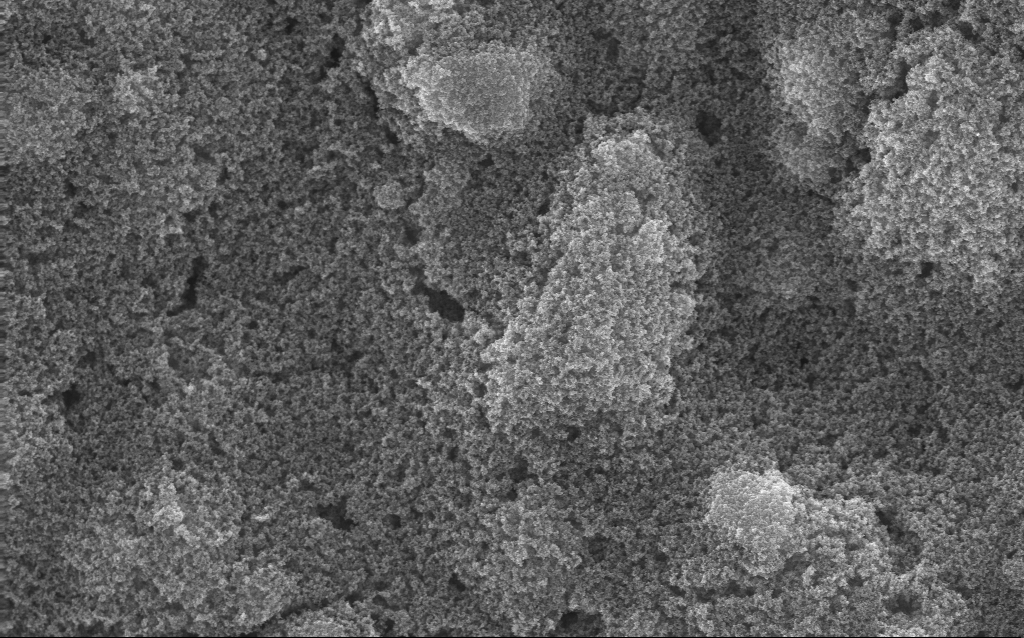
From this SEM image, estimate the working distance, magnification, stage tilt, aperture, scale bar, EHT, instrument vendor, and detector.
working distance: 2.8 mm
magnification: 23.9 K X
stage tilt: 0°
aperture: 30 µm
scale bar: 1000 nm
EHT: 10 kV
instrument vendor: Zeiss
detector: InLens